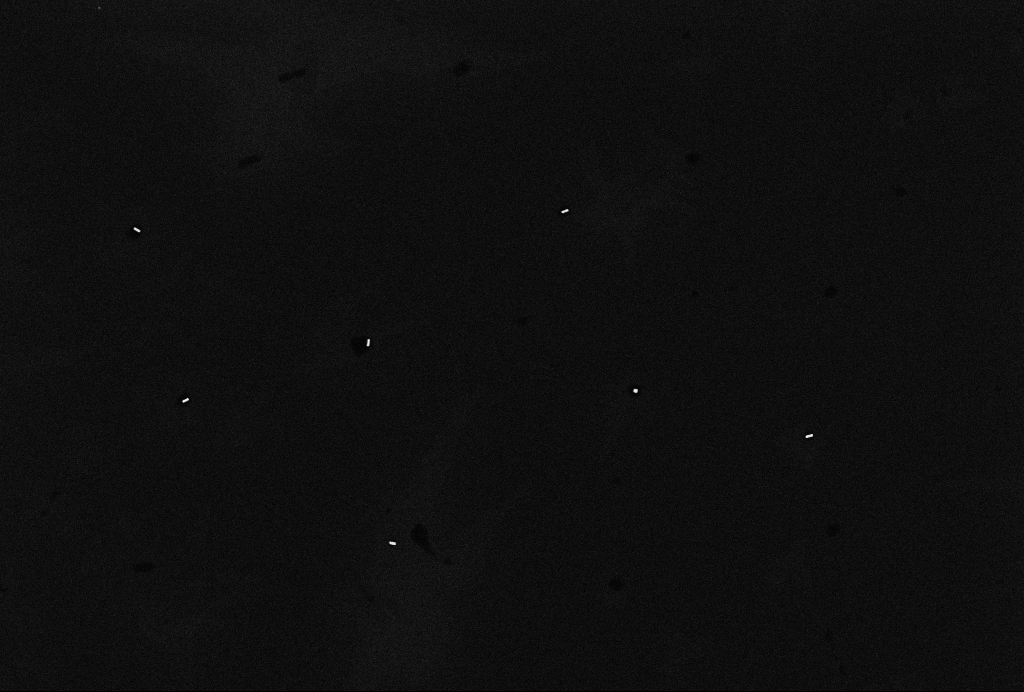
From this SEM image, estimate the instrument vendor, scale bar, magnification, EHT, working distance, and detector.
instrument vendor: Zeiss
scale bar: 1000 nm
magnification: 35.36 K X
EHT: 10 kV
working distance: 3.2 mm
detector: InLens